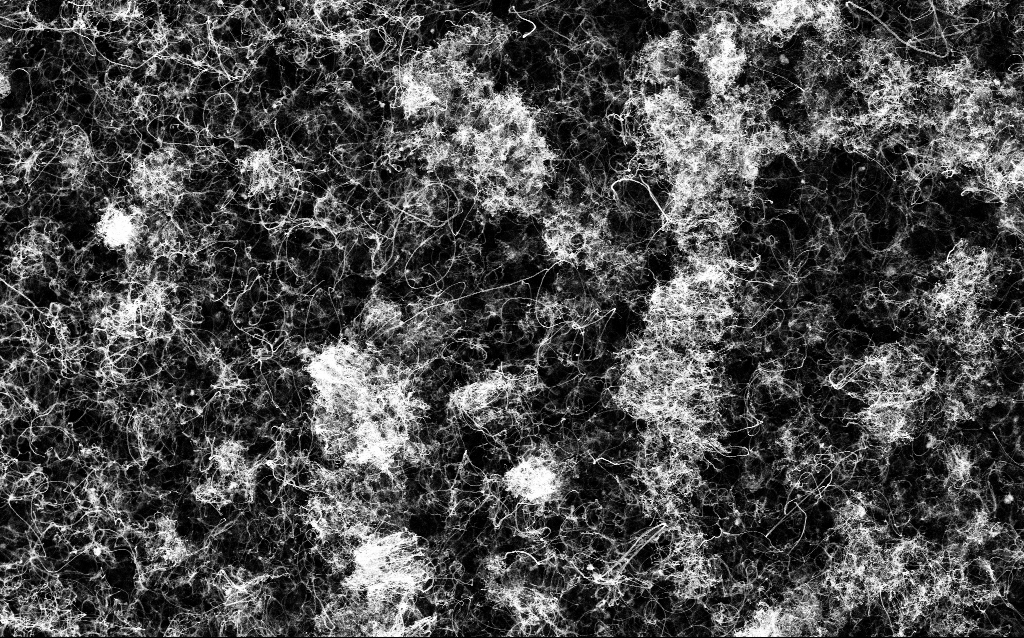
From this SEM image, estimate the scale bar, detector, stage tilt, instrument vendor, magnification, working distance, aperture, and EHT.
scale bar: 1000 nm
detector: InLens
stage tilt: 0°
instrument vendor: Zeiss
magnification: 20 K X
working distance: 3.3 mm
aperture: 30 µm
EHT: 5 kV